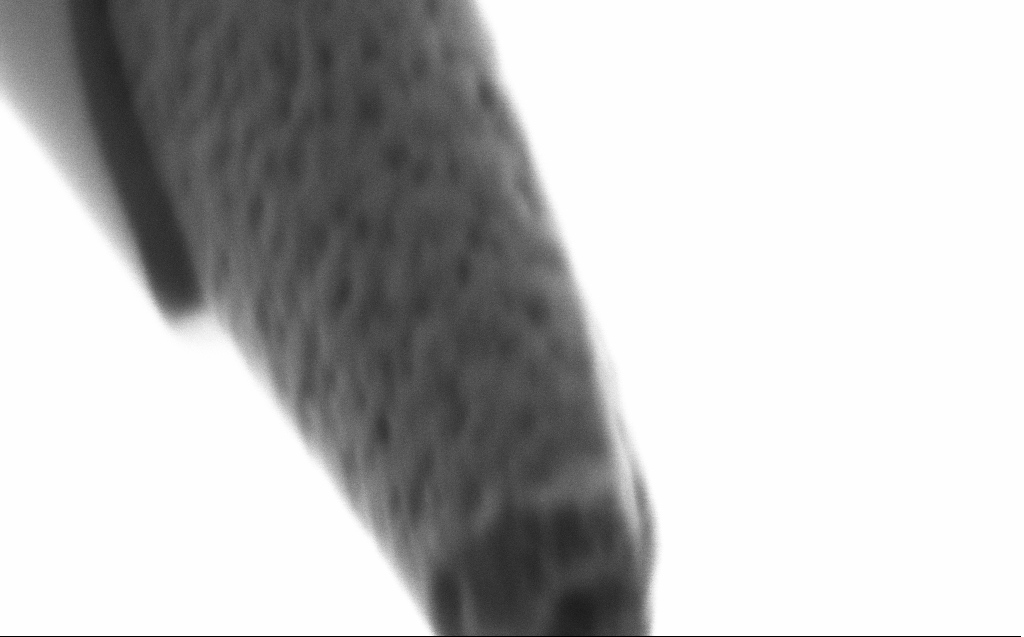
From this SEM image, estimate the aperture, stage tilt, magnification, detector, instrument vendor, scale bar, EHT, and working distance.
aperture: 30 µm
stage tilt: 45°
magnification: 250 K X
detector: SE2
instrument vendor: Zeiss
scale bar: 200 nm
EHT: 0.8 kV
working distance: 4 mm